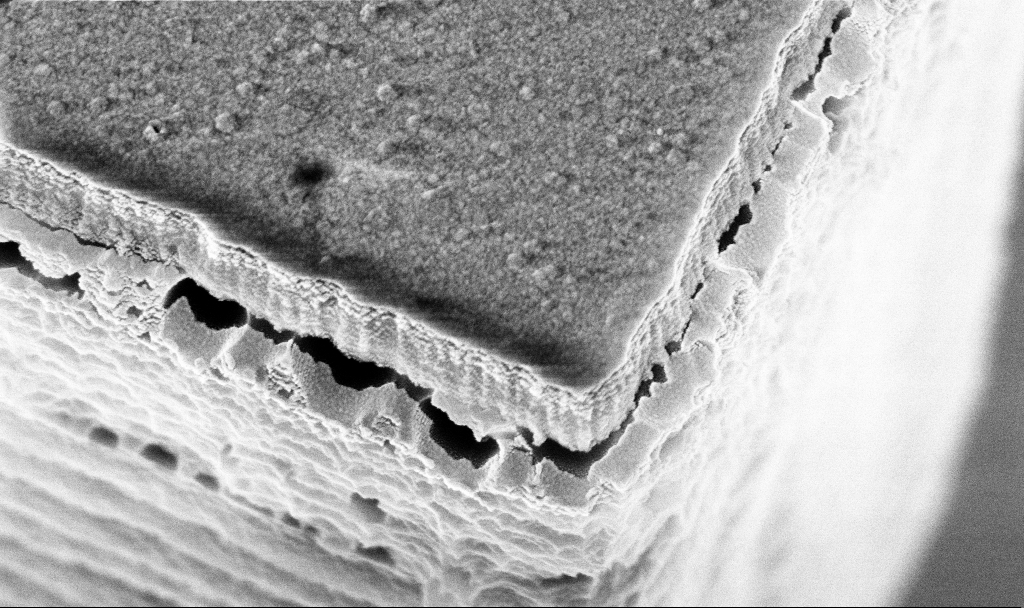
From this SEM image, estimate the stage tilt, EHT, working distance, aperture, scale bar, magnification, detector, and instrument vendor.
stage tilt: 19.9°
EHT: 5 kV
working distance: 3.1 mm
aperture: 30 µm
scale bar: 200 nm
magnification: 108.24 K X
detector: InLens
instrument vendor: Zeiss